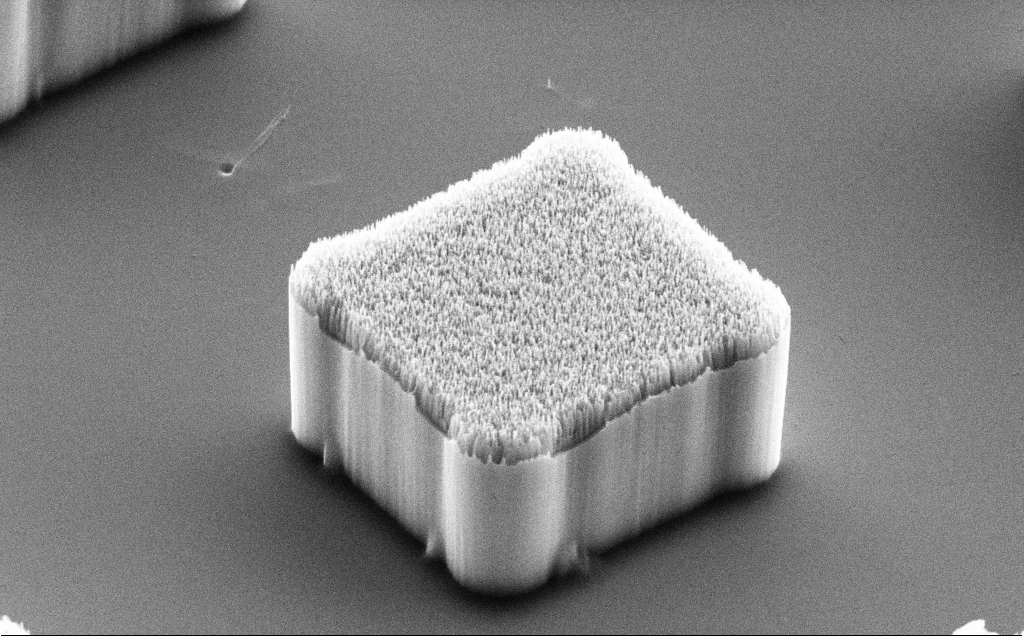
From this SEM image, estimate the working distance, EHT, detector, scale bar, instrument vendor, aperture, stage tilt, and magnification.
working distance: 13 mm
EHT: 10 kV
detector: SE2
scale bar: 2000 nm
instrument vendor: Zeiss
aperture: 30 µm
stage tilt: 50.6°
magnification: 16.74 K X